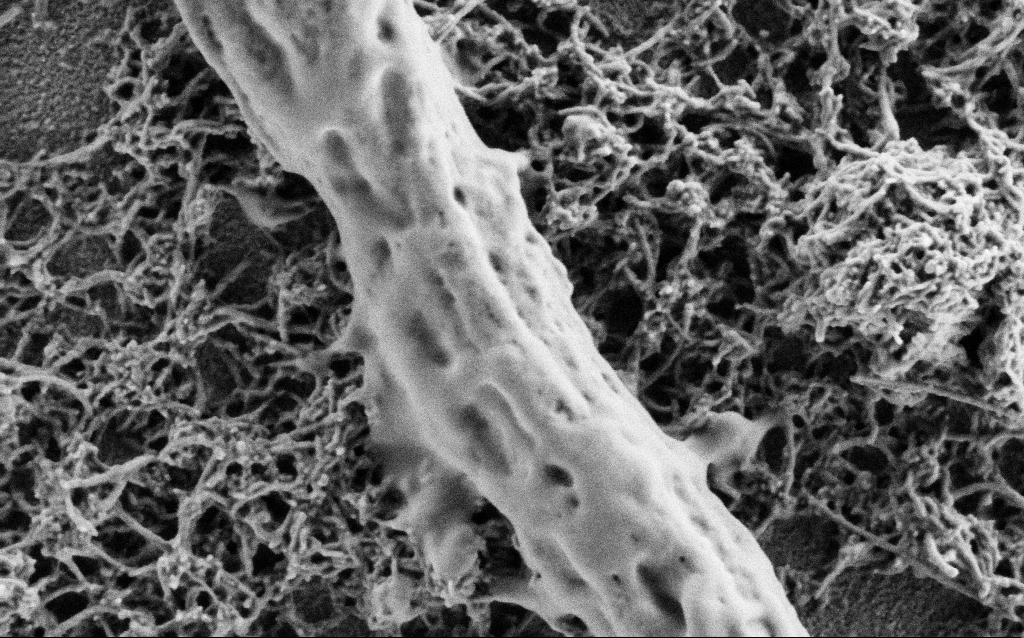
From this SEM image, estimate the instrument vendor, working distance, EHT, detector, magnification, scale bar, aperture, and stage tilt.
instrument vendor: Zeiss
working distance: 4 mm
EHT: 1 kV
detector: SE2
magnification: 75 K X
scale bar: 200 nm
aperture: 30 µm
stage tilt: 0°